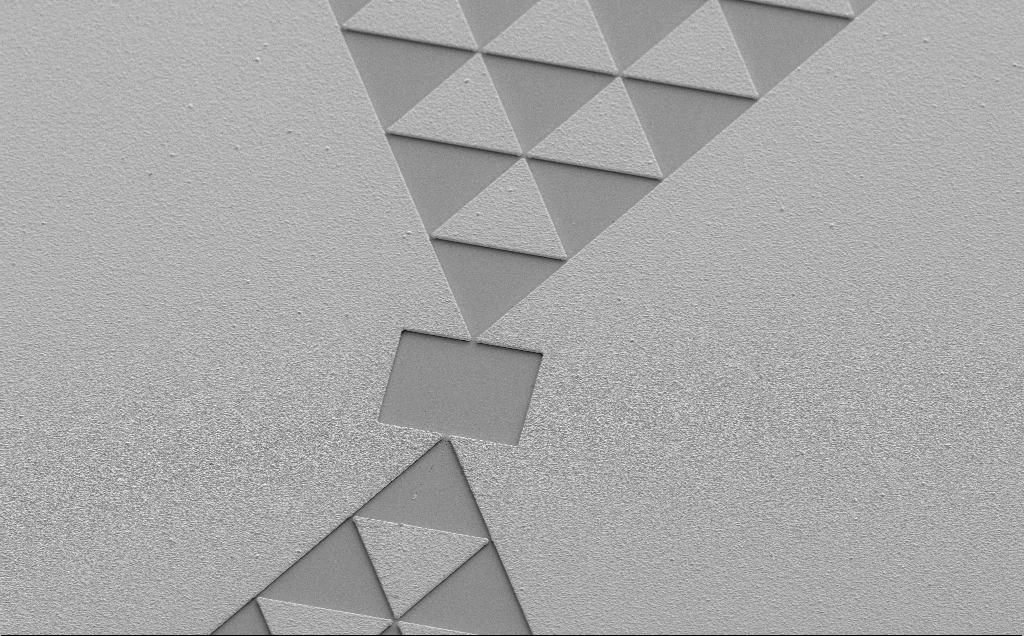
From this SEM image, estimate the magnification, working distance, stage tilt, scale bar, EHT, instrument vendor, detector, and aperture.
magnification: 0.879 K X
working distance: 13 mm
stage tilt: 35°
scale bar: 20000 nm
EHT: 5 kV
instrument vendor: Zeiss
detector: SE2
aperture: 30 µm